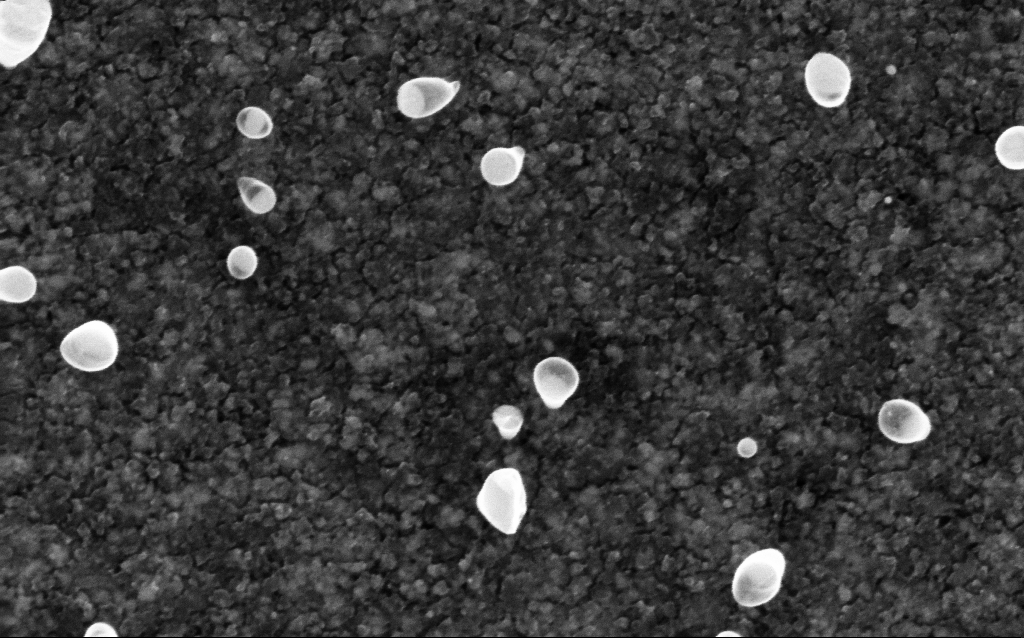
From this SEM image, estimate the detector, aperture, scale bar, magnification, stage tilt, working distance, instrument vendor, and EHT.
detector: InLens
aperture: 30 µm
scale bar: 100 nm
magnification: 200 K X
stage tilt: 0°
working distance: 2.1 mm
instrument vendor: Zeiss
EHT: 5 kV